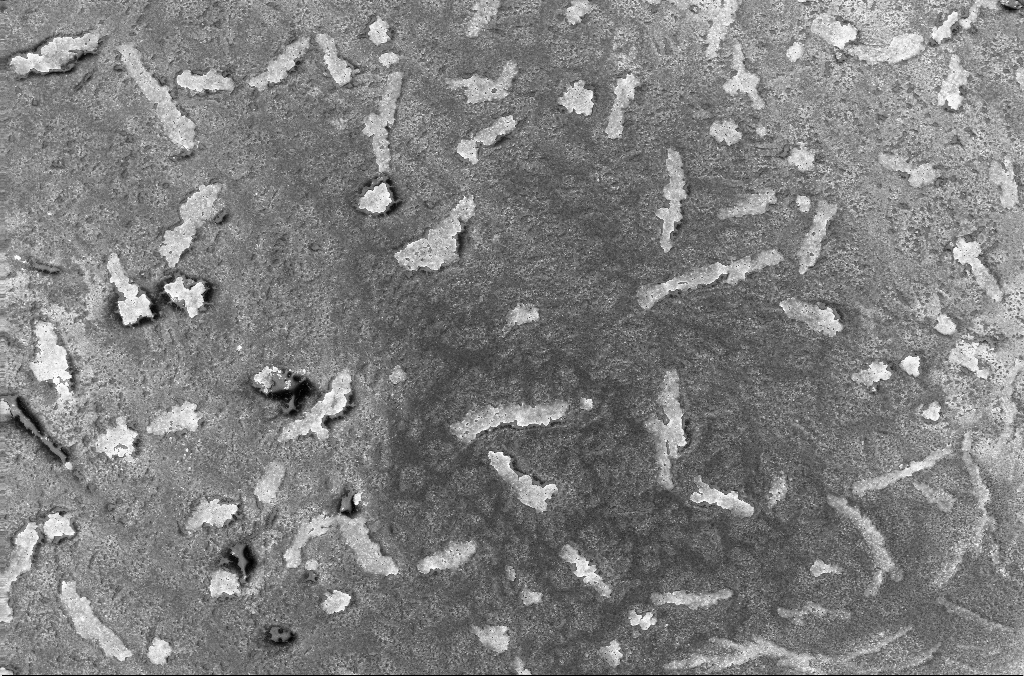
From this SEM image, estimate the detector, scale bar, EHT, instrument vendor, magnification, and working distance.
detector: InLens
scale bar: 100000 nm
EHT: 20 kV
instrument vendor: Zeiss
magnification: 0.5 K X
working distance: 2.7 mm